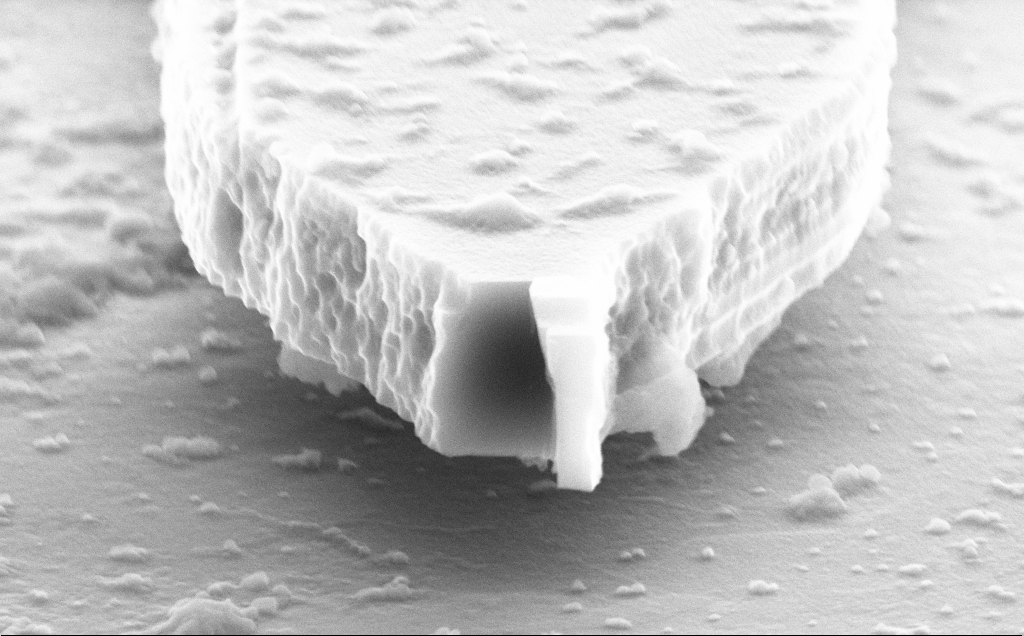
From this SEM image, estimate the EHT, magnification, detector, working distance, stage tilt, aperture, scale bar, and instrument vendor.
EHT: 10 kV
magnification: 28.46 K X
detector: SE2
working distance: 9 mm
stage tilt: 70°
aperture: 30 µm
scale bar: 2000 nm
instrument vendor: Zeiss